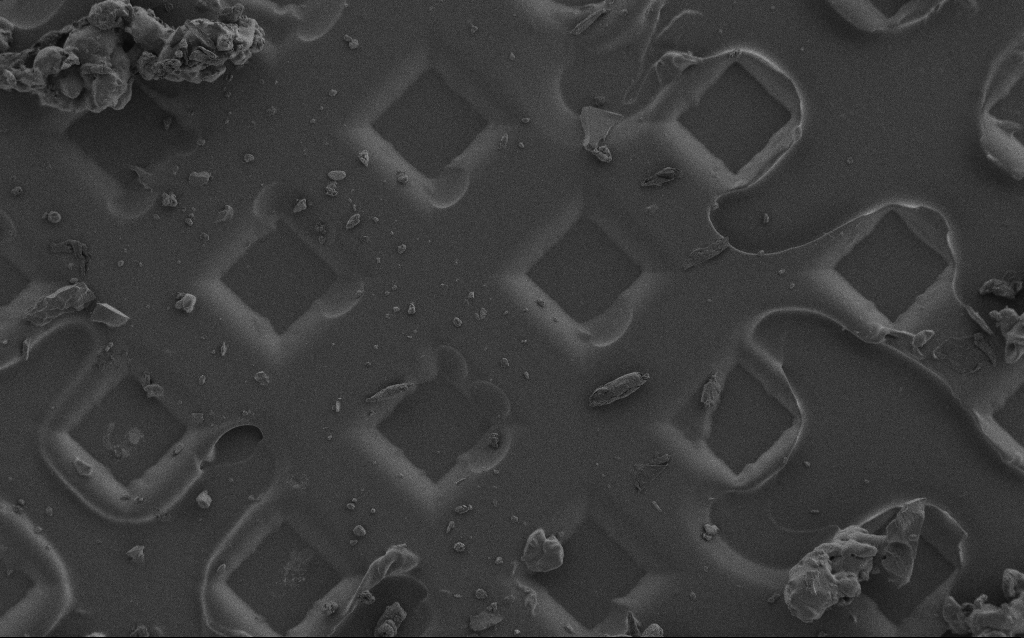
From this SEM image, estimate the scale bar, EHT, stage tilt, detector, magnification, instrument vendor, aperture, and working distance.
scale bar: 100000 nm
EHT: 1.5 kV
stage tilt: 0°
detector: SE2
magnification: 0.553 K X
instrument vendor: Zeiss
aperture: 30 µm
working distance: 7 mm